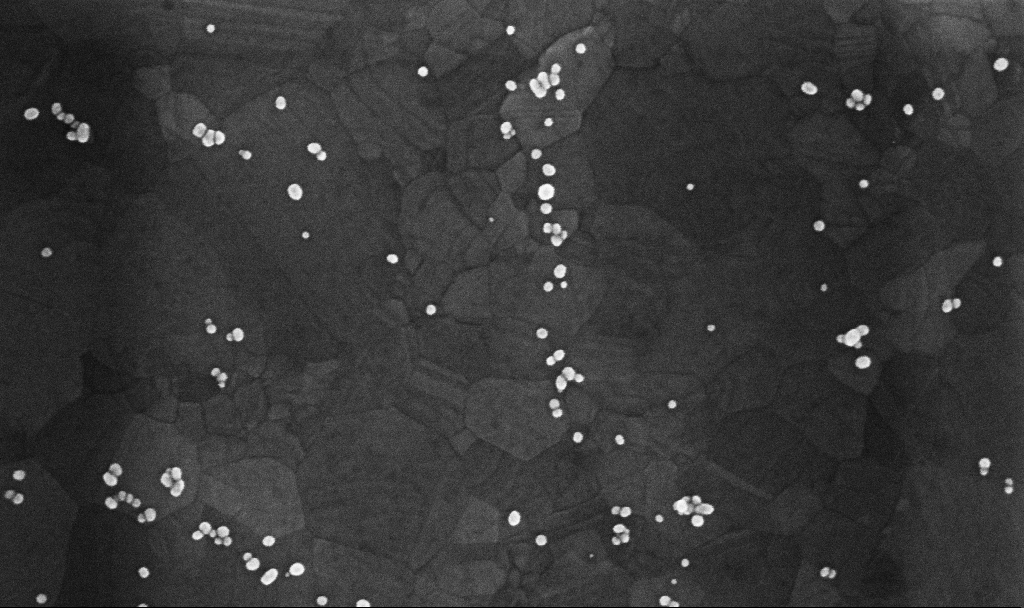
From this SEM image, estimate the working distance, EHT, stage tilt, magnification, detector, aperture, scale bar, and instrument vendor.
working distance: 3.4 mm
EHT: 10 kV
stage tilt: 0°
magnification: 179.42 K X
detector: InLens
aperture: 30 µm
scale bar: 200 nm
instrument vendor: Zeiss